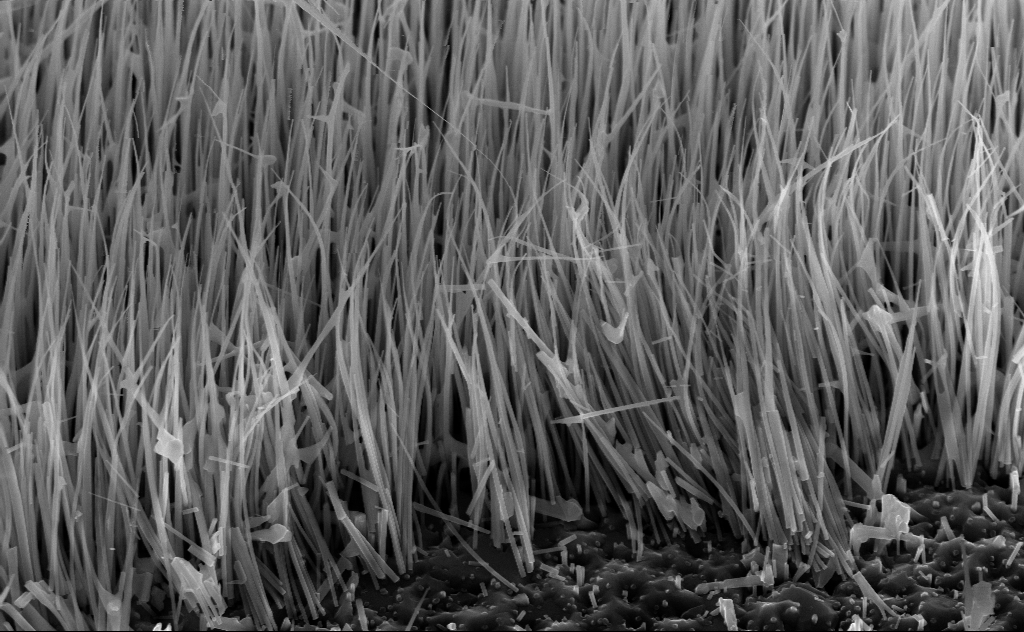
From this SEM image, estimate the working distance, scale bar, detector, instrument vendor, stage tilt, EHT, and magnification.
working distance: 6 mm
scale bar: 1000 nm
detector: InLens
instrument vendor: Zeiss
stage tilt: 45°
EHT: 10 kV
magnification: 40 K X